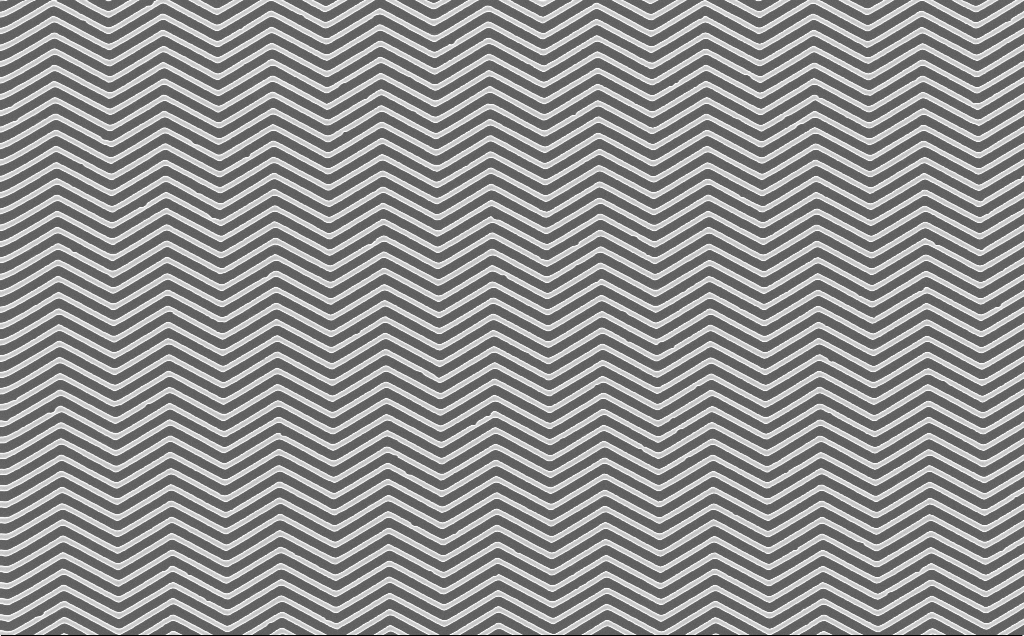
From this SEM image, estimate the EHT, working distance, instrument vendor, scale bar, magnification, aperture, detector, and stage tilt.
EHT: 10 kV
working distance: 4 mm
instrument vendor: Zeiss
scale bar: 1000 nm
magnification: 20 K X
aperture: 30 µm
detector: InLens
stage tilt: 0°